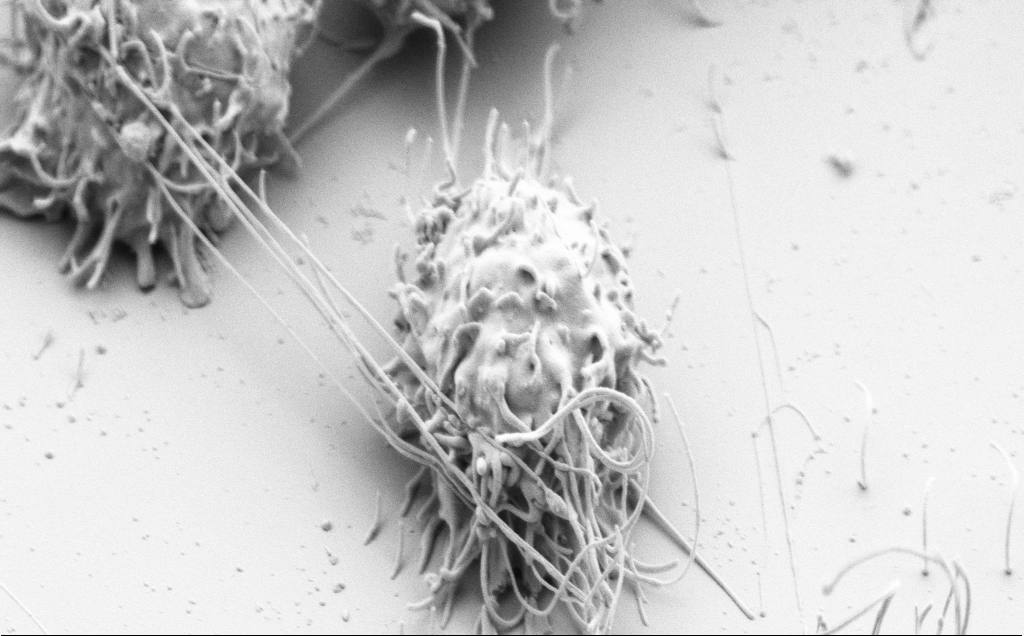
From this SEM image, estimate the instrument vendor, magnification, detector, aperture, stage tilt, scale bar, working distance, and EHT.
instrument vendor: Zeiss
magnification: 12.5 K X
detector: SE2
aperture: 30 µm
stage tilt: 46.1°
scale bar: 2000 nm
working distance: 7 mm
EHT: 3 kV